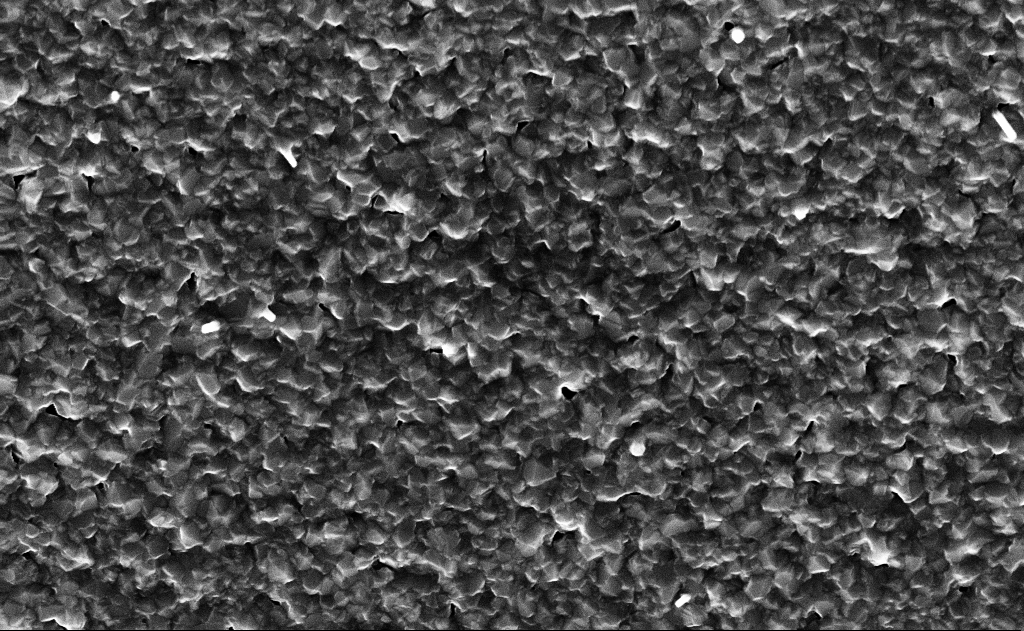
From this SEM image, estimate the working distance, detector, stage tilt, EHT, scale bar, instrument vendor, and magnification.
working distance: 11 mm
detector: InLens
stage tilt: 0°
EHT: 10 kV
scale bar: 1000 nm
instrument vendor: Zeiss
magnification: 40 K X